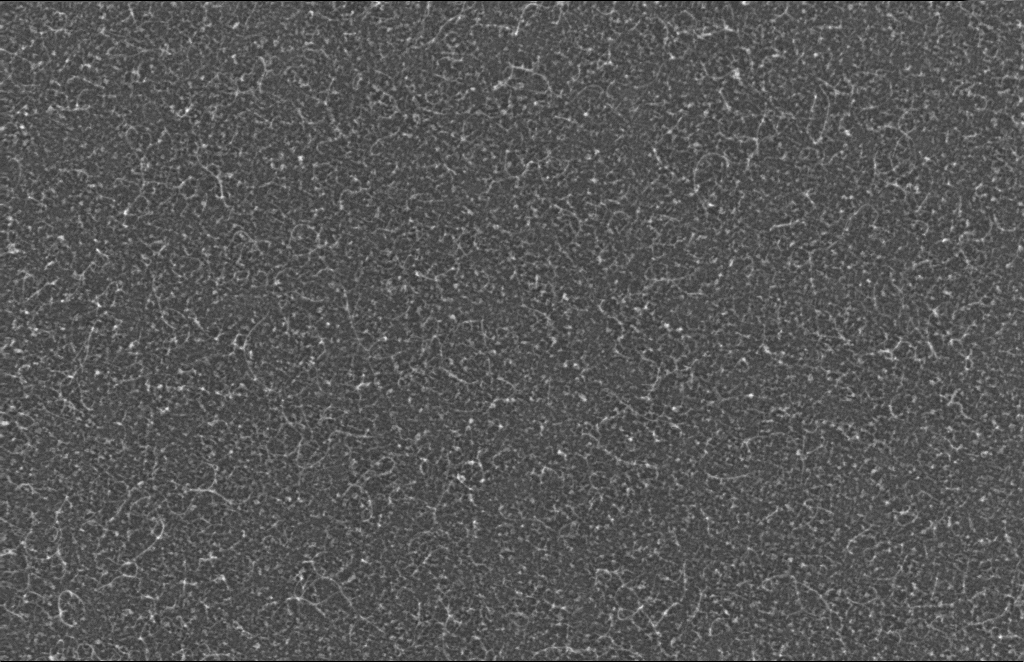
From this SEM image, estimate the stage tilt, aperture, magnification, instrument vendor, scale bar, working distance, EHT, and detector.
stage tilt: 0°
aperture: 30 µm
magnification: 135.3 K X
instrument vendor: Zeiss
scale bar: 200 nm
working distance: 6 mm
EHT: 5 kV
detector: InLens